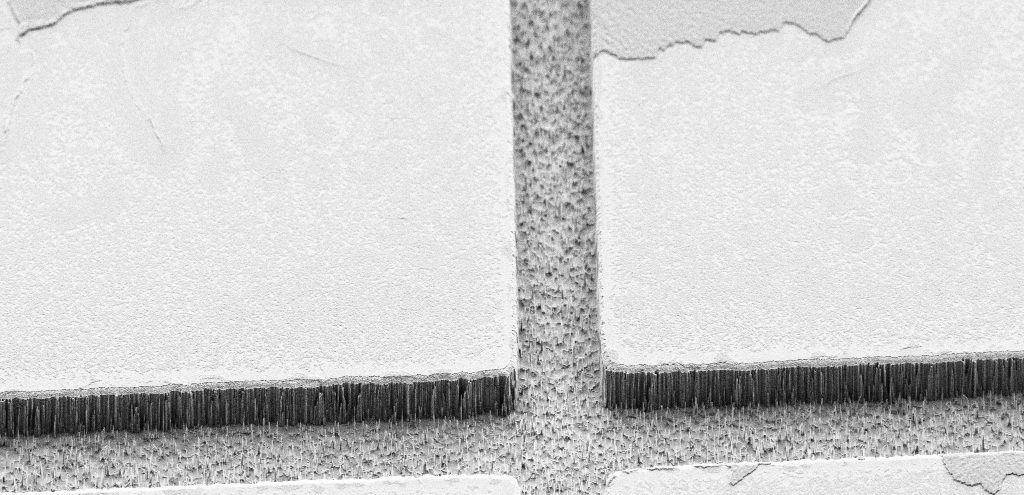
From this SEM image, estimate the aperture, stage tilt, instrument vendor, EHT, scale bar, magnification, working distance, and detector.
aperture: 30 µm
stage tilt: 45°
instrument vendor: Zeiss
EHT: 2 kV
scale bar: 10000 nm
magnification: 2.82 K X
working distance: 8 mm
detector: SE2